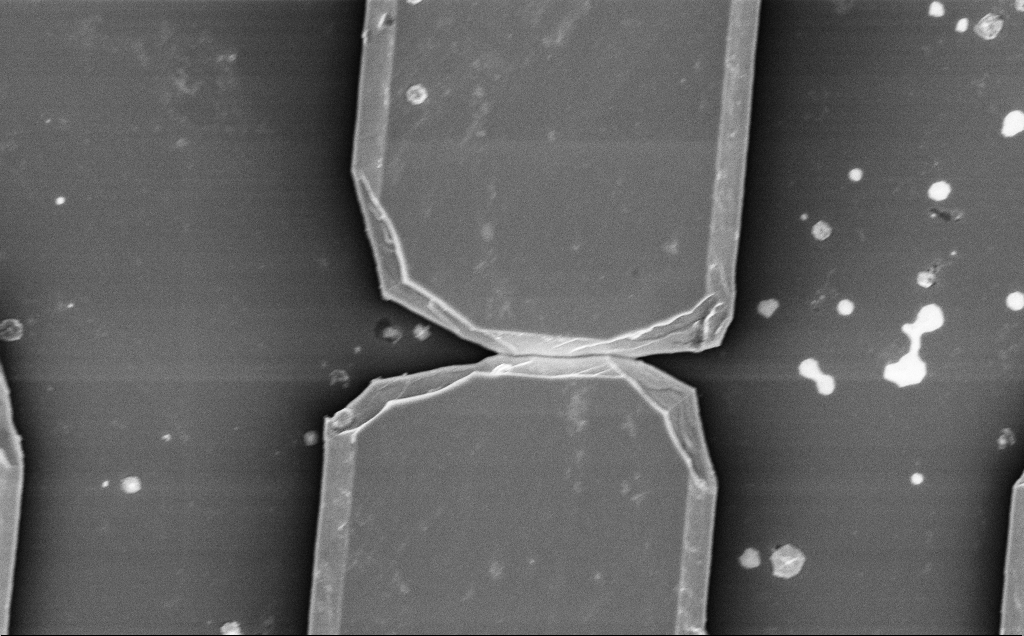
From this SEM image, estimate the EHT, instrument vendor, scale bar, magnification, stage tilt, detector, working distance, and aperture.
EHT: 5 kV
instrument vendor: Zeiss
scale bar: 10000 nm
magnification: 6.33 K X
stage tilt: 0°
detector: InLens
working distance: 14 mm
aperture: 30 µm